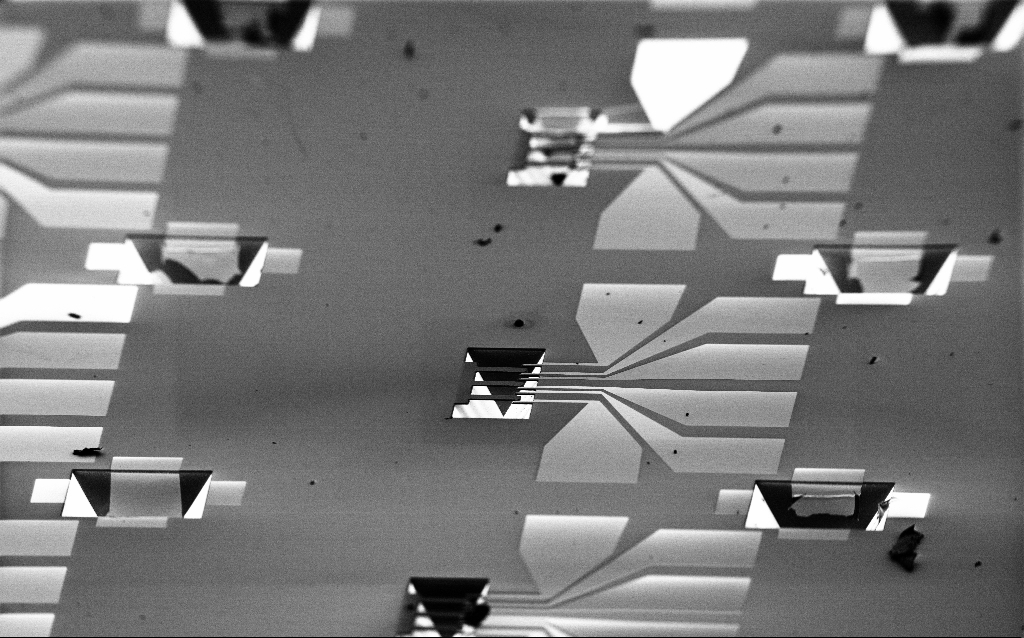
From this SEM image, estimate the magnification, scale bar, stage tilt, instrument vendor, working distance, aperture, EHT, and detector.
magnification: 0.249 K X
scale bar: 100000 nm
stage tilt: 70°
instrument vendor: Zeiss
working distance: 8.9 mm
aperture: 30 µm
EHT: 2 kV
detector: SE2